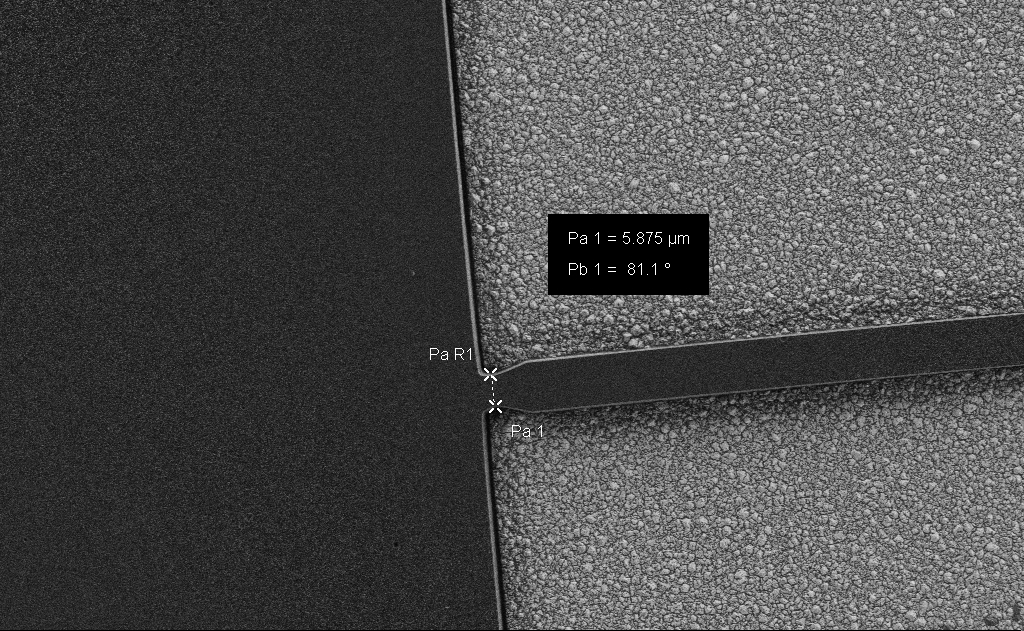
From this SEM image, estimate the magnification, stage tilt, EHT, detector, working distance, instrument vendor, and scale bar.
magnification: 2.02 K X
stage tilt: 0°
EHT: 1.2 kV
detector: SE2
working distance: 5 mm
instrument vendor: Zeiss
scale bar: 20000 nm